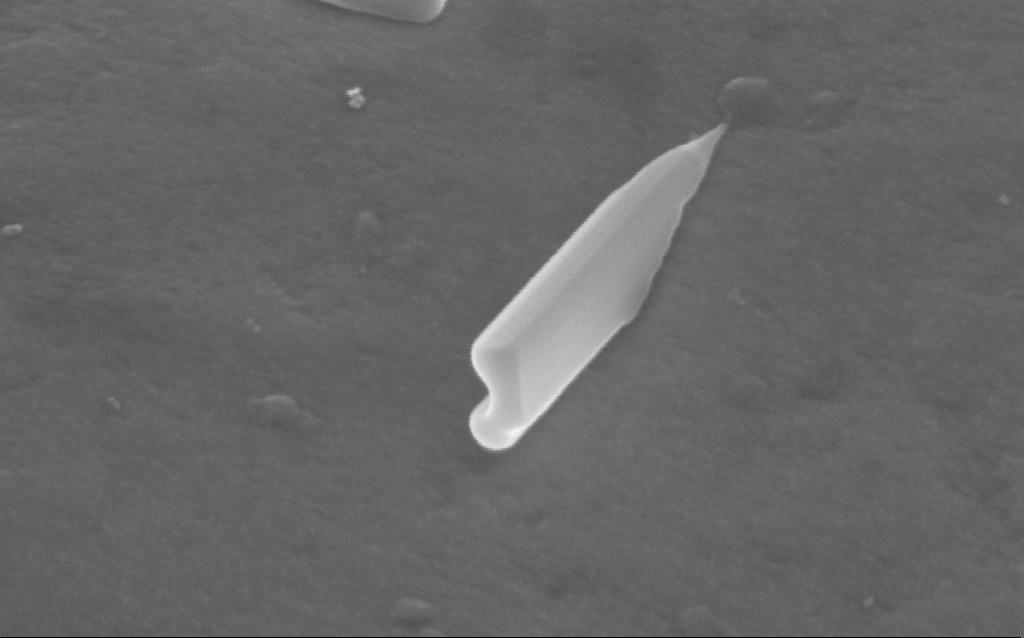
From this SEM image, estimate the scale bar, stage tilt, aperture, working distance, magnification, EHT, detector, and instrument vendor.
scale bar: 200 nm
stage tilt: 35°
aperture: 30 µm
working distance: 4 mm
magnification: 187.4 K X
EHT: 5 kV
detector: InLens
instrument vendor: Zeiss